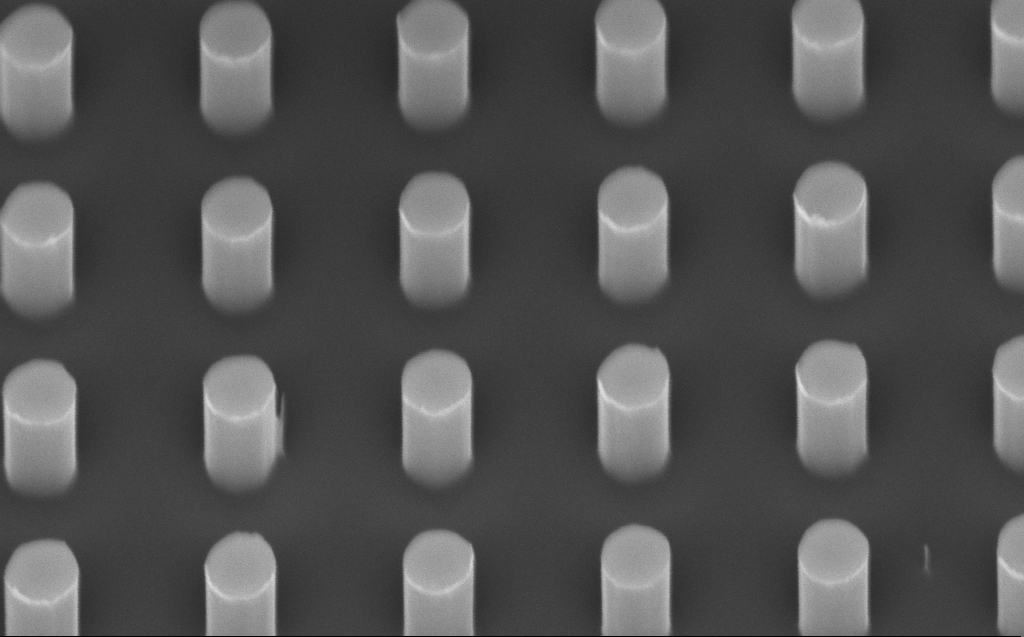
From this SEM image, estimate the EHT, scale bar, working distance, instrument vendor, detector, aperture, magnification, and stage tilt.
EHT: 5 kV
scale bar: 1000 nm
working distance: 6 mm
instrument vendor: Zeiss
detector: InLens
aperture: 30 µm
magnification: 69.4 K X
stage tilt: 45°